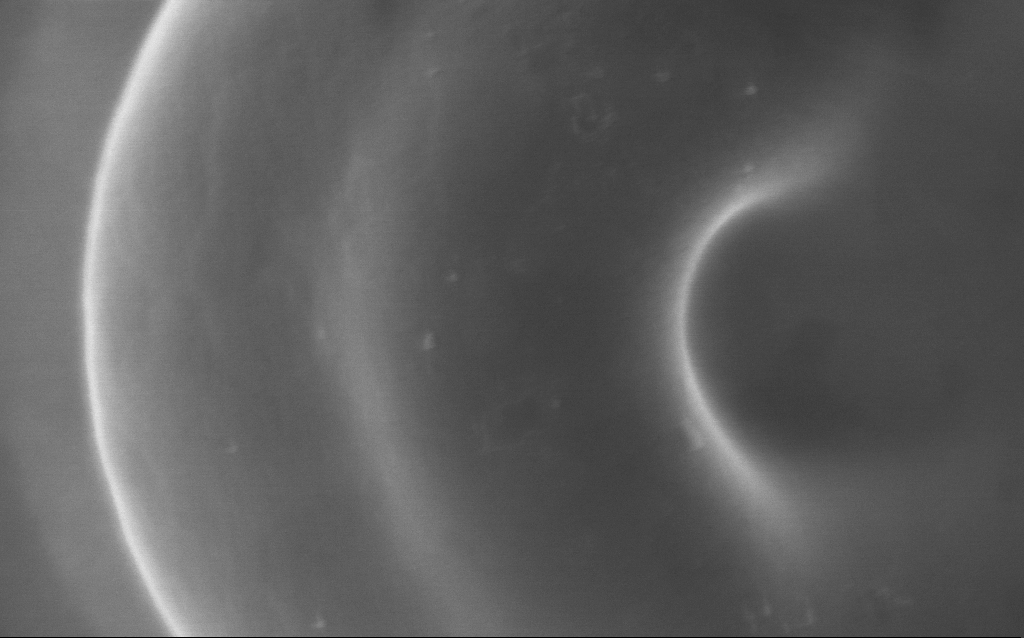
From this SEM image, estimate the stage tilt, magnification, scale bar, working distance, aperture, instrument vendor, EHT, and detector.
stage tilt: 0°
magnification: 136 K X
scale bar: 200 nm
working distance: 3 mm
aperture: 30 µm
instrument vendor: Zeiss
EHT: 5 kV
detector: InLens